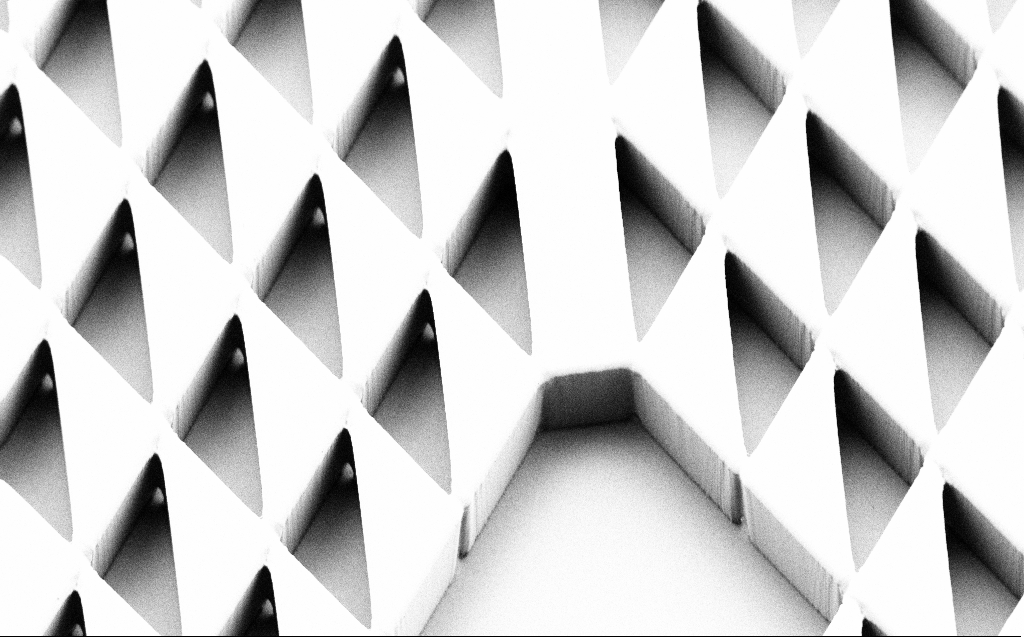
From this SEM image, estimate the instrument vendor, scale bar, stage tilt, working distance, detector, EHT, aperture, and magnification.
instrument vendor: Zeiss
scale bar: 20000 nm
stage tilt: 45°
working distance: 6 mm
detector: SE2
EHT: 1 kV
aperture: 30 µm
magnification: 0.989 K X